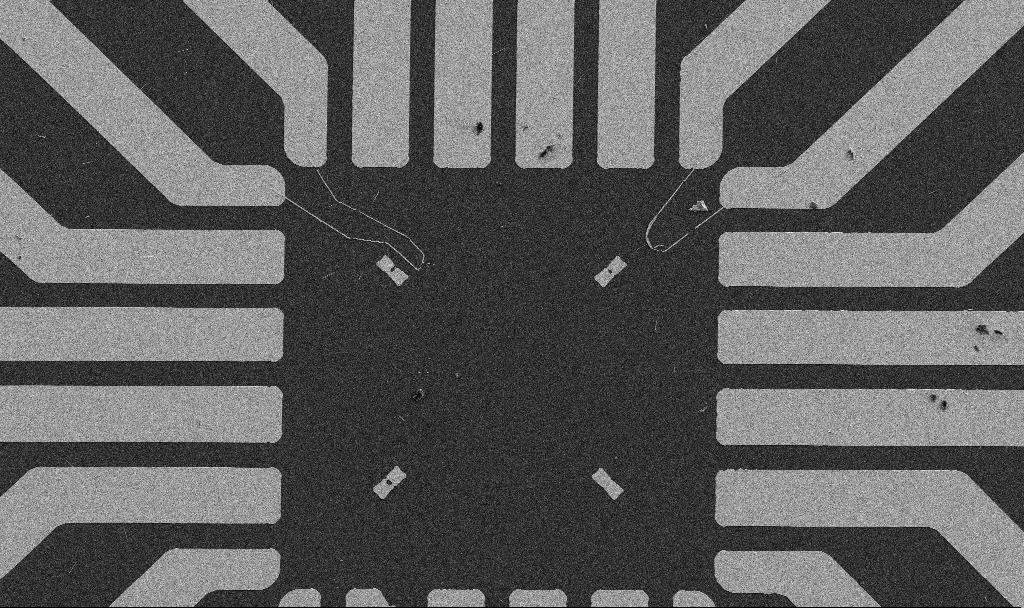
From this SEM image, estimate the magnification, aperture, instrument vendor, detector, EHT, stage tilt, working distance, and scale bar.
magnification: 1 K X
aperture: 30 µm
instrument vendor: Zeiss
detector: SE2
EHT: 5 kV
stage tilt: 0°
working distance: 10.7 mm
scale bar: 20000 nm